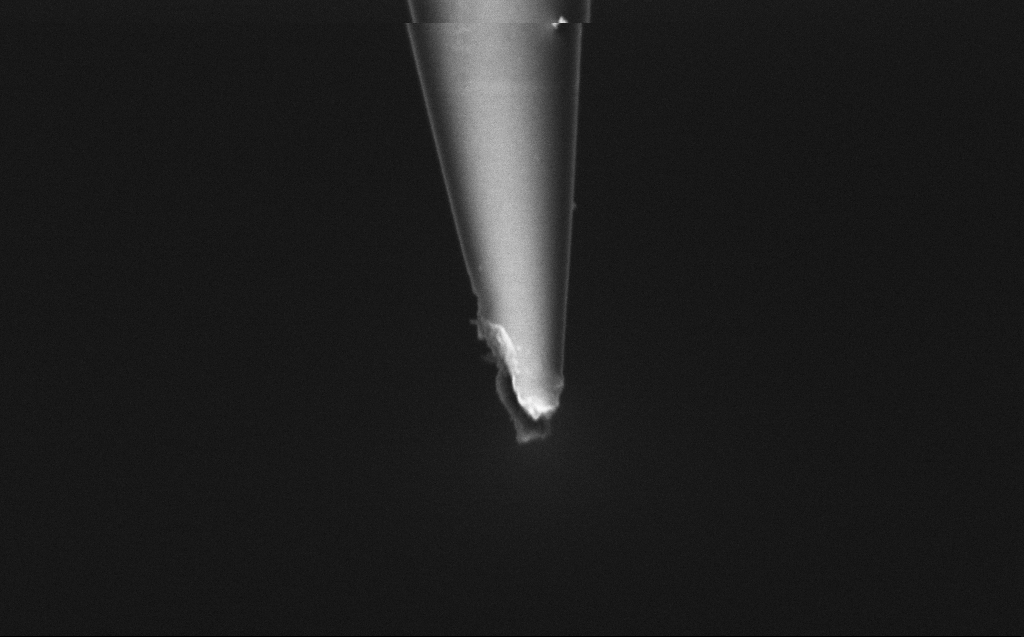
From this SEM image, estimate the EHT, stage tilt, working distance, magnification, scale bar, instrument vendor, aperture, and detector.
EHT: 2 kV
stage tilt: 45°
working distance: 6 mm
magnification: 100 K X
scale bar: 200 nm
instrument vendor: Zeiss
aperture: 30 µm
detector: InLens